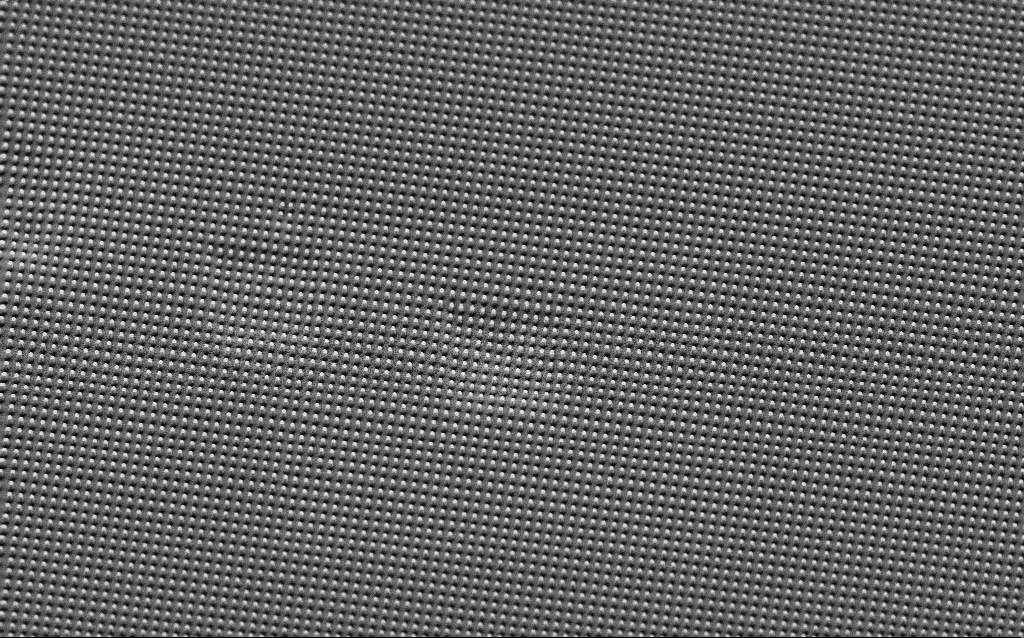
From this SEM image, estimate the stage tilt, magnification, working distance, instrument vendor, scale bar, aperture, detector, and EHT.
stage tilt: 45°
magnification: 7.98 K X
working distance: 5.7 mm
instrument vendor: Zeiss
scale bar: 2000 nm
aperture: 30 µm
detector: SE2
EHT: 3 kV